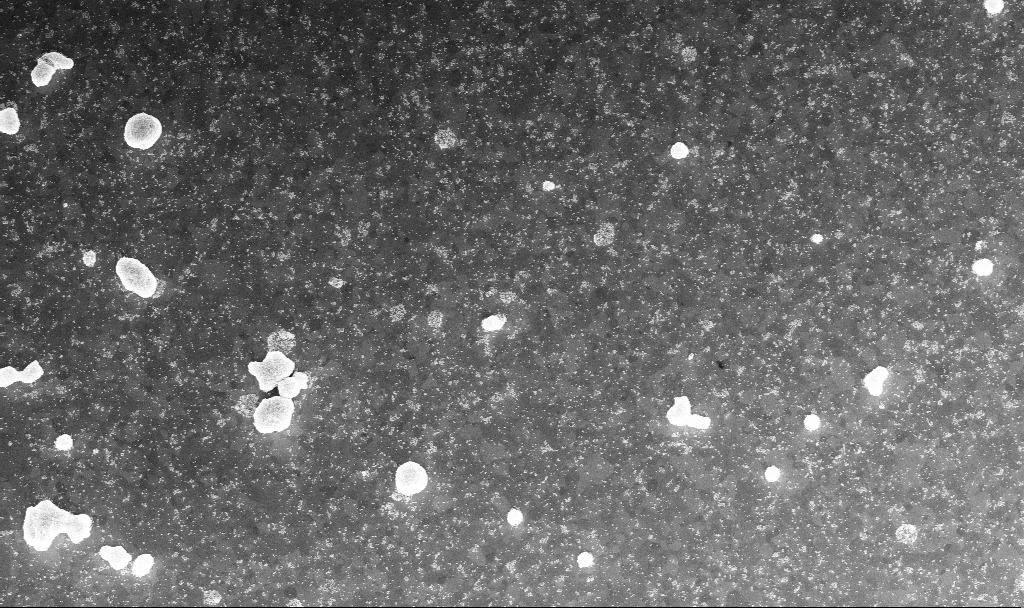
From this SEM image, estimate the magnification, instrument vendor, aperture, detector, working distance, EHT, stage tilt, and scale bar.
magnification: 20.58 K X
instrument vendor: Zeiss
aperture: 30 µm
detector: InLens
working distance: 3.9 mm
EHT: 10 kV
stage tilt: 0°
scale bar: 1000 nm